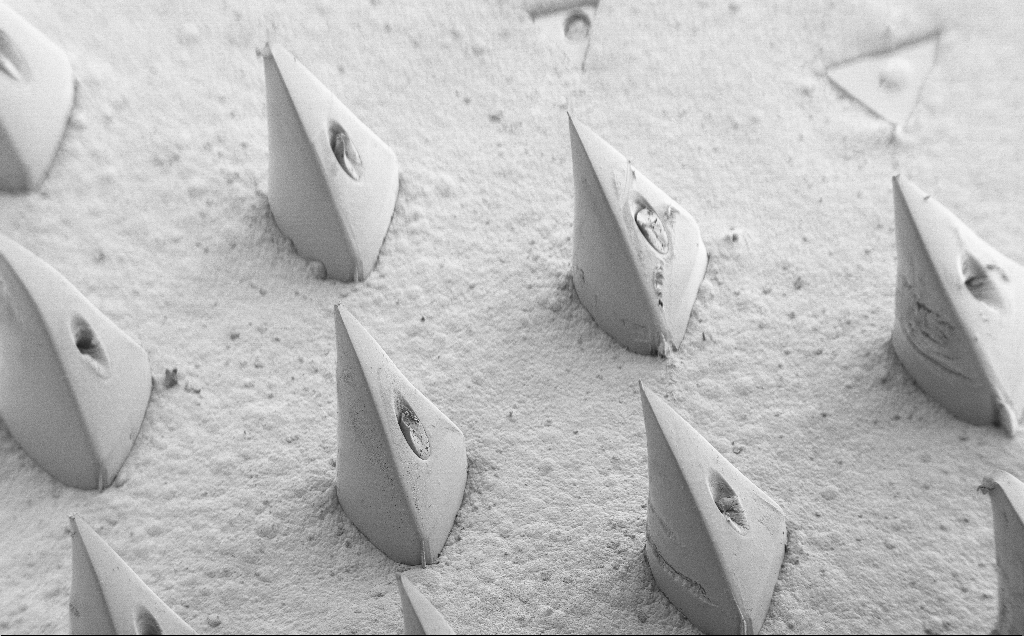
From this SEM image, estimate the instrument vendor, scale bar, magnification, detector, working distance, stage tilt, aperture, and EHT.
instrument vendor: Zeiss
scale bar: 200000 nm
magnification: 0.081 K X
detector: SE2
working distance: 8 mm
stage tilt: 40°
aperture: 30 µm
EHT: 5 kV